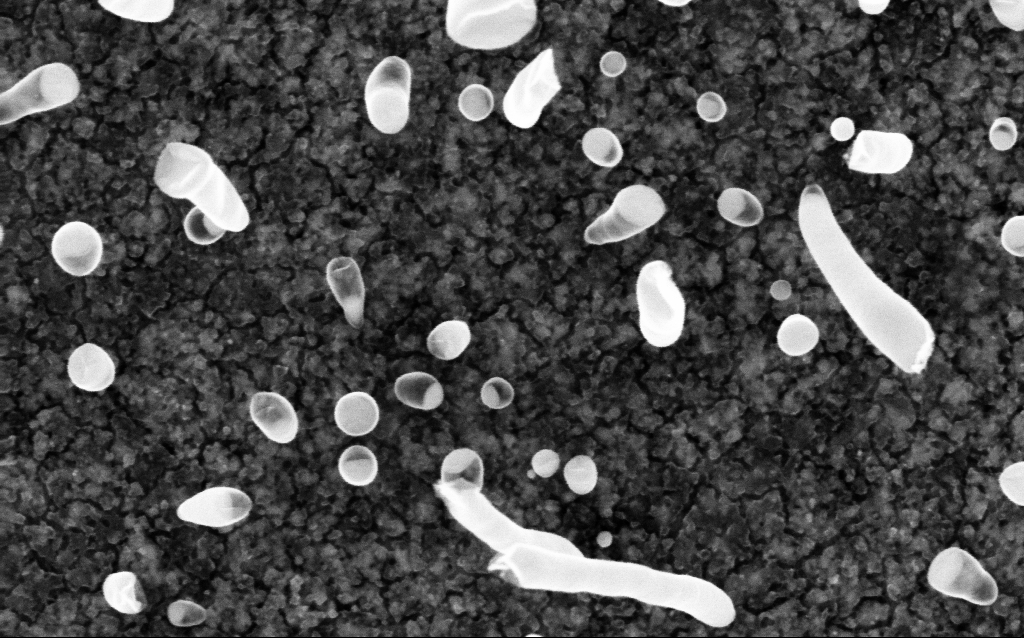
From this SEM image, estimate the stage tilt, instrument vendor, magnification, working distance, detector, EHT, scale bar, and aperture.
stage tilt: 0°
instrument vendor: Zeiss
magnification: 200 K X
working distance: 2 mm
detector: InLens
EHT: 5 kV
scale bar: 100 nm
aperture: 30 µm